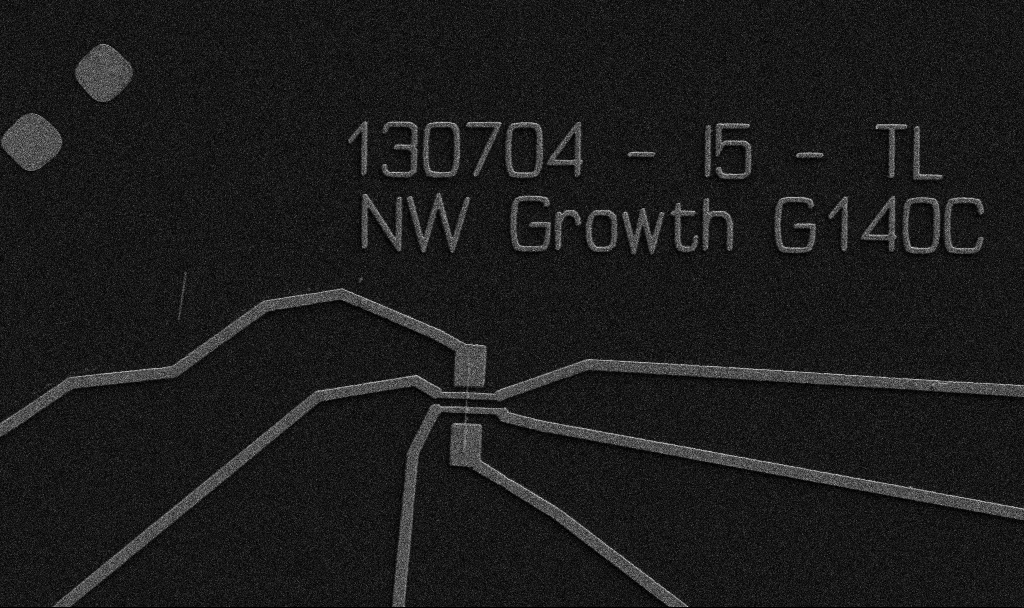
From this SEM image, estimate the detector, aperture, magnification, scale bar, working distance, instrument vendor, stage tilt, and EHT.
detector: SE2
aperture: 30 µm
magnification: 5 K X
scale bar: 10000 nm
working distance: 10.7 mm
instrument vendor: Zeiss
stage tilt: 0°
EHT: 5 kV